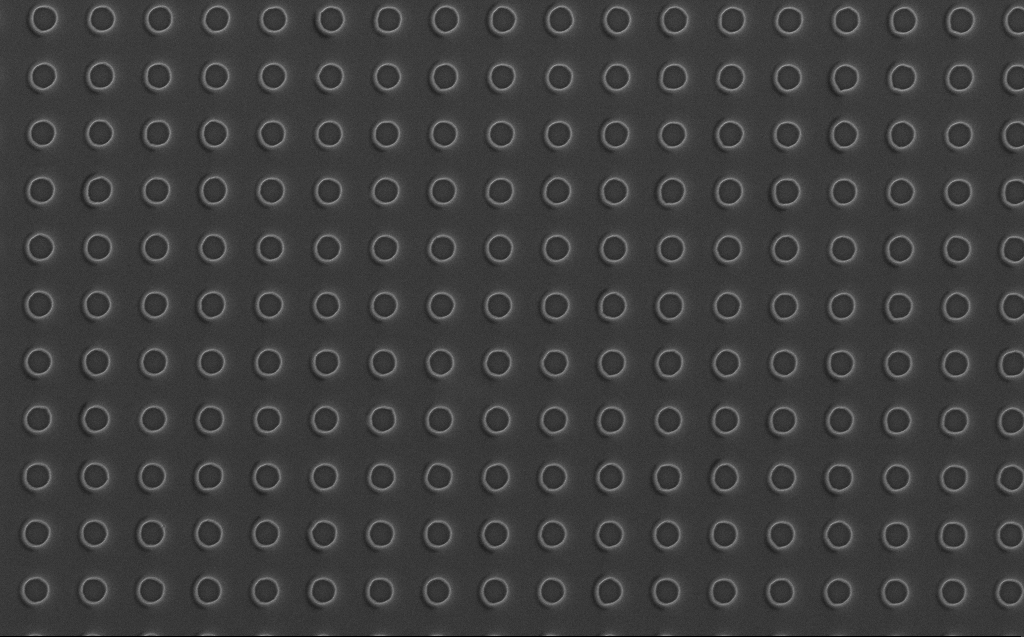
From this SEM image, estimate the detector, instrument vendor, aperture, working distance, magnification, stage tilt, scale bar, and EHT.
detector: InLens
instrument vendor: Zeiss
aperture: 30 µm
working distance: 7 mm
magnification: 20 K X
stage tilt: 0°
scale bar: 2000 nm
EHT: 5 kV